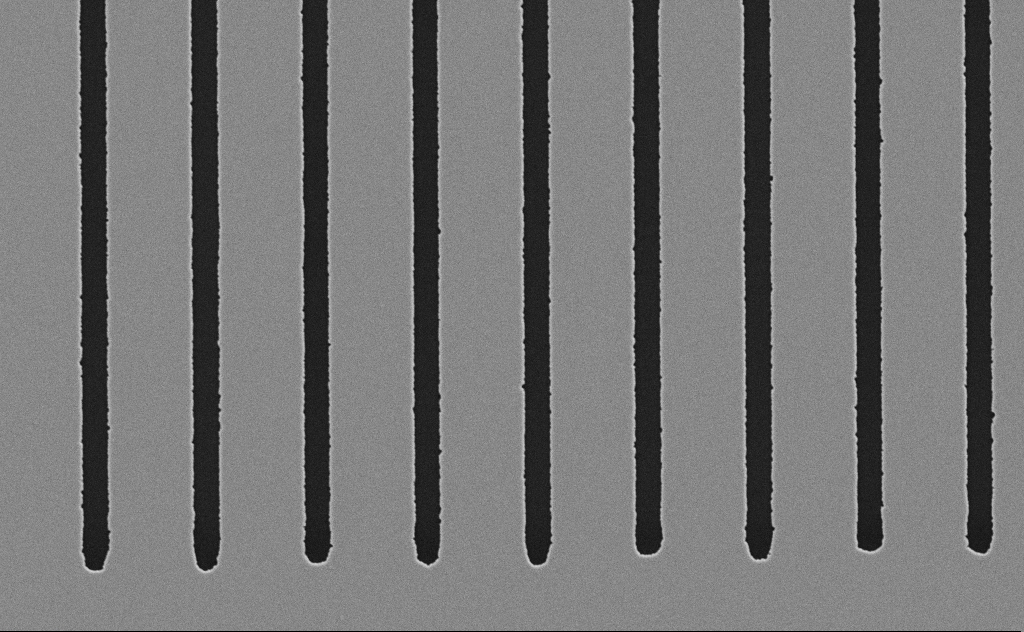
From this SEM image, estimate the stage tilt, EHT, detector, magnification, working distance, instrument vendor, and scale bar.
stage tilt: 0°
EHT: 5 kV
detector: SE2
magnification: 10.15 K X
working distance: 8 mm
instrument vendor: Zeiss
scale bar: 2000 nm